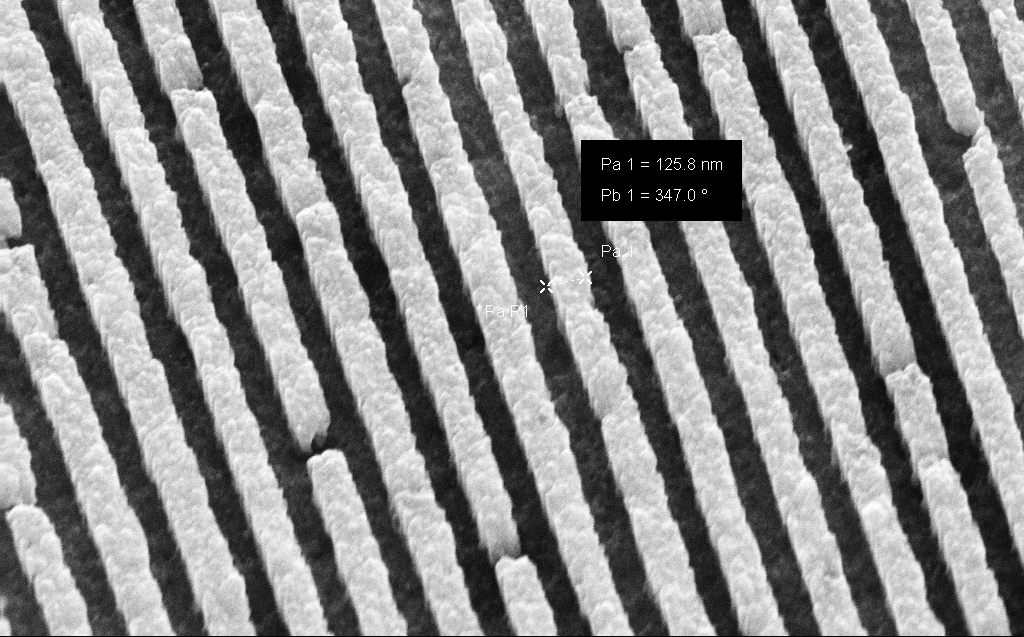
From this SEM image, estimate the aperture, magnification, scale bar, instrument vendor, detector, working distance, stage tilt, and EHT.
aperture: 30 µm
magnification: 116.86 K X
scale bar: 200 nm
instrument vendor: Zeiss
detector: SE2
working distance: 5 mm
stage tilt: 45°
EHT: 30 kV